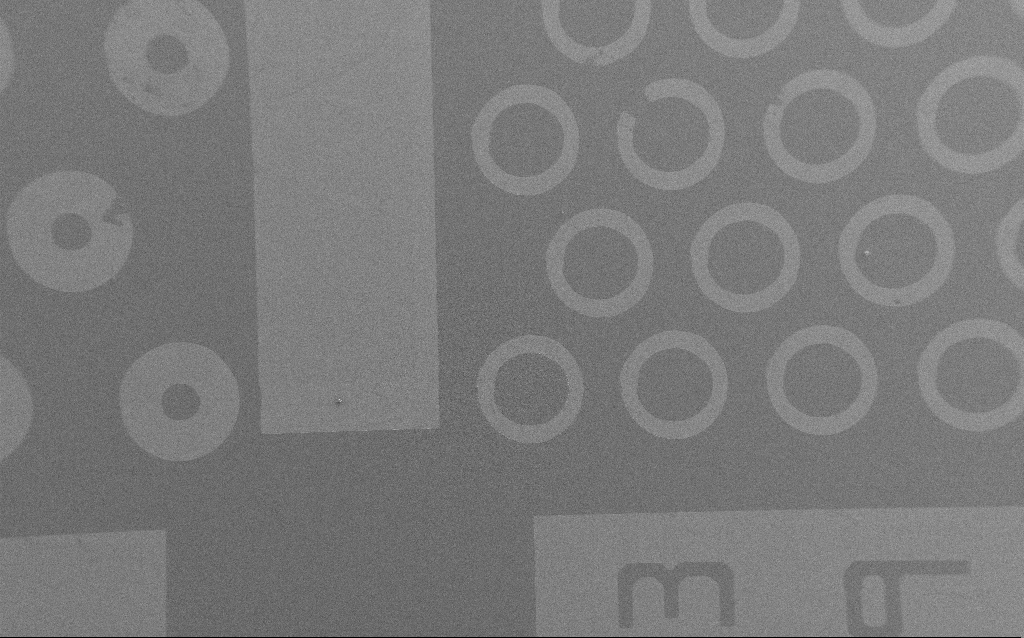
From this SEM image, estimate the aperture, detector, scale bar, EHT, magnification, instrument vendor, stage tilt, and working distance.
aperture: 30 µm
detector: SE2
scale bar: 100000 nm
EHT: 2 kV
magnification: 0.167 K X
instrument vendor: Zeiss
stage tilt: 0°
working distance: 4 mm